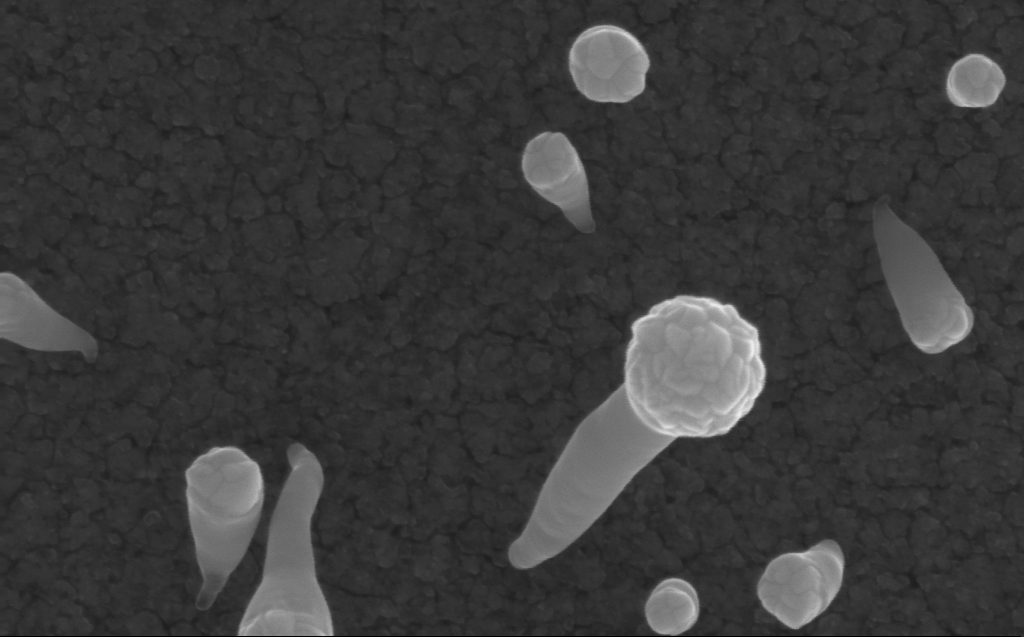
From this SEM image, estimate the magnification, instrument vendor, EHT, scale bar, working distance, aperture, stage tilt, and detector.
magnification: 200 K X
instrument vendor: Zeiss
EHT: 10 kV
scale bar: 100 nm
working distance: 3 mm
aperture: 30 µm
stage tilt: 0°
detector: InLens